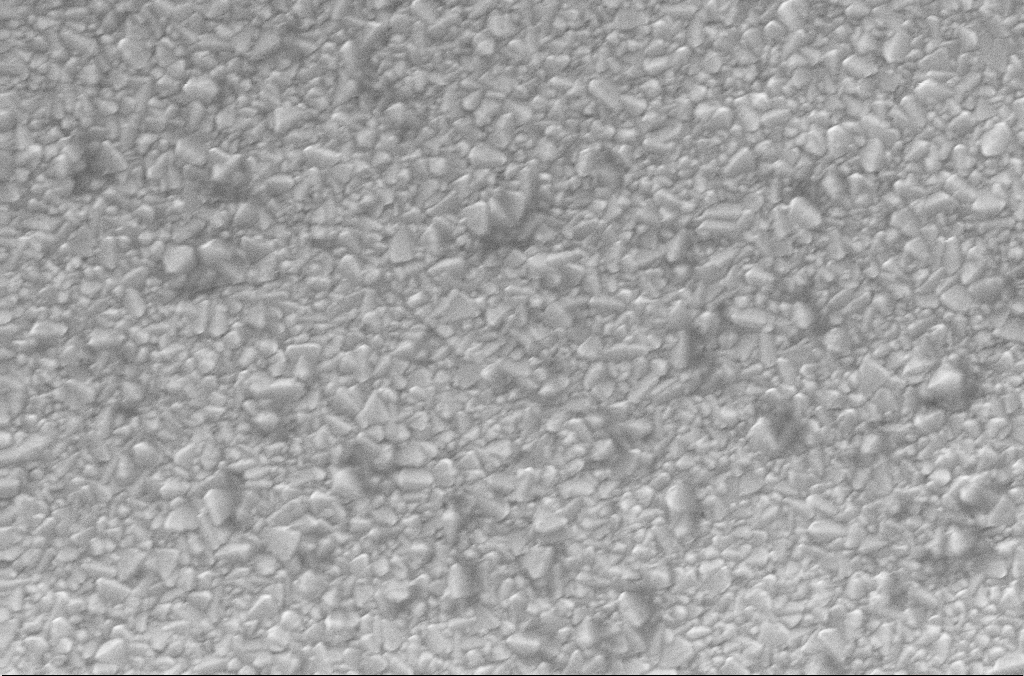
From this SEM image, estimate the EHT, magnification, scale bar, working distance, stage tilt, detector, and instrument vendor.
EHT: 20 kV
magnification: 30 K X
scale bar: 2000 nm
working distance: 1.9 mm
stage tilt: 0°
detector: InLens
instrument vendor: Zeiss